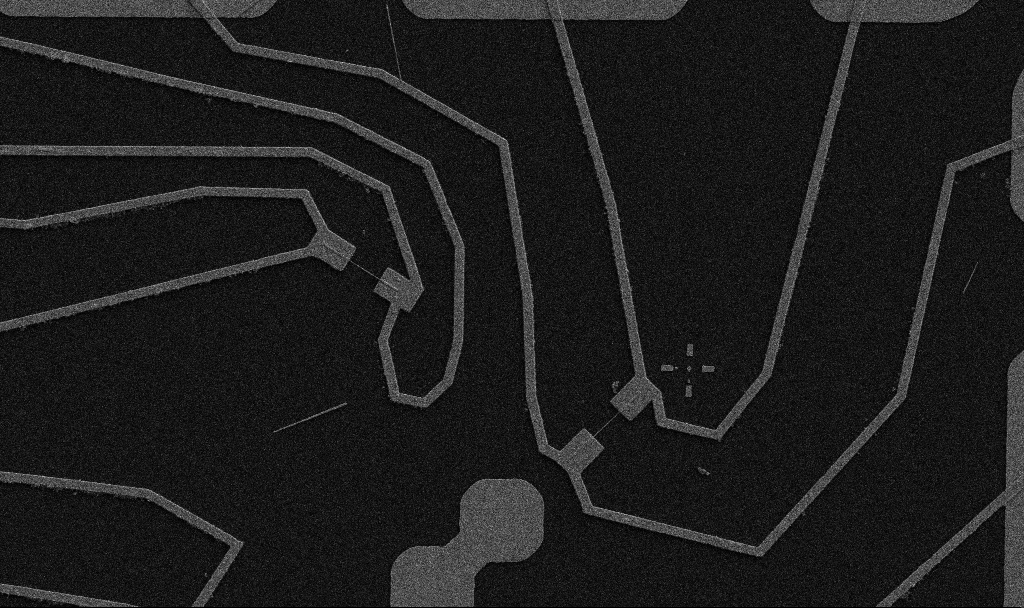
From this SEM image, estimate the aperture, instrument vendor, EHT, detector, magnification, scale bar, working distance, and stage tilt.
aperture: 30 µm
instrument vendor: Zeiss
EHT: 5 kV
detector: SE2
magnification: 5 K X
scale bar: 10000 nm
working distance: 10.7 mm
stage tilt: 0°